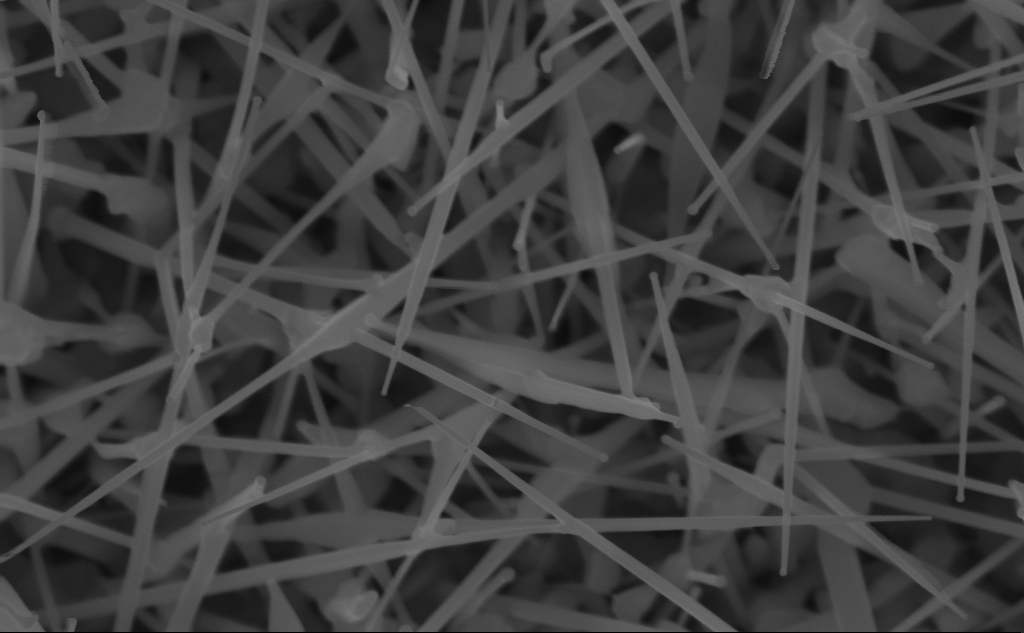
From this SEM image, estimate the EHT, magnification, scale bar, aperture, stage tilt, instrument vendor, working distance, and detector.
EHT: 10 kV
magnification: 80 K X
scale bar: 200 nm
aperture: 30 µm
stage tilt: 0°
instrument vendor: Zeiss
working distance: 5 mm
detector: InLens